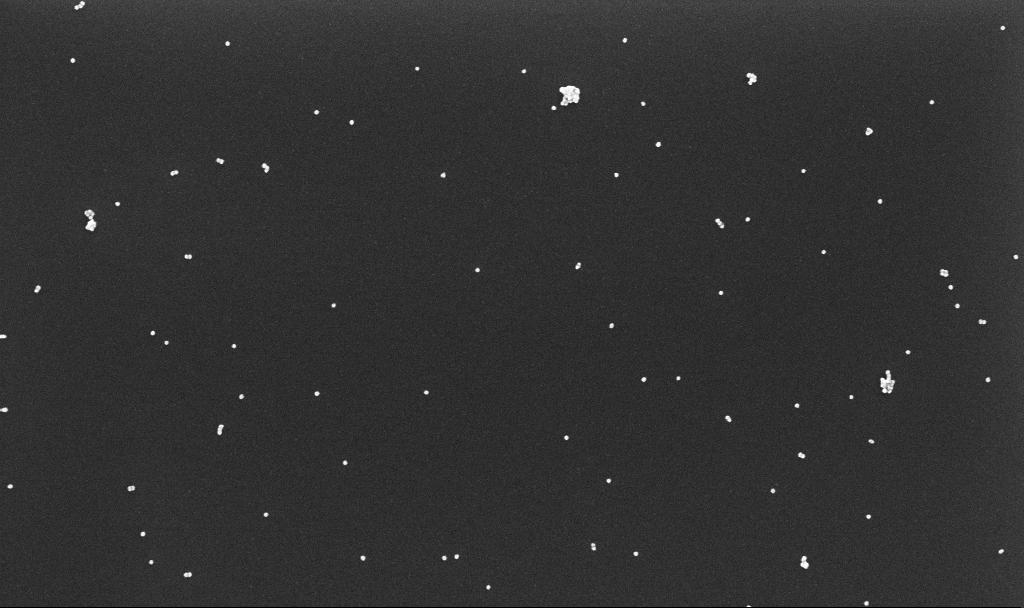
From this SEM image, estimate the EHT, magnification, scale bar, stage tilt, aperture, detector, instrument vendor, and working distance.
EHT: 10 kV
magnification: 70 K X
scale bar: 1000 nm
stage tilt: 0°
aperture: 30 µm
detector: InLens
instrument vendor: Zeiss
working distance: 3.4 mm